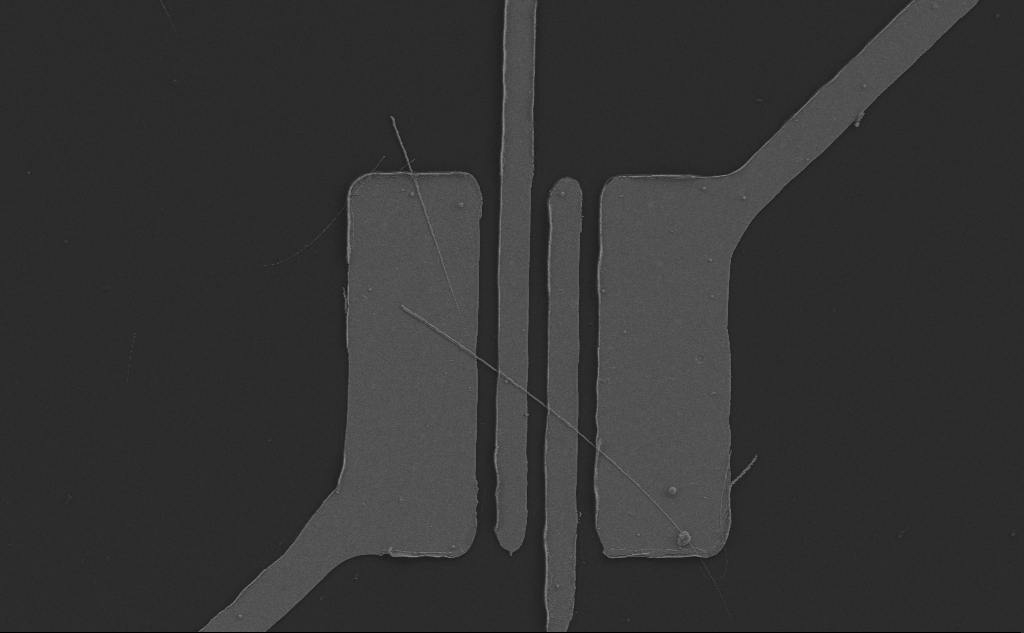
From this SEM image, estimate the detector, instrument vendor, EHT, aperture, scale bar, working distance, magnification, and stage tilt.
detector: SE2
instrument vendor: Zeiss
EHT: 5 kV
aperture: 30 µm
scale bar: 10000 nm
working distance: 6 mm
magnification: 4.64 K X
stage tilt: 0°